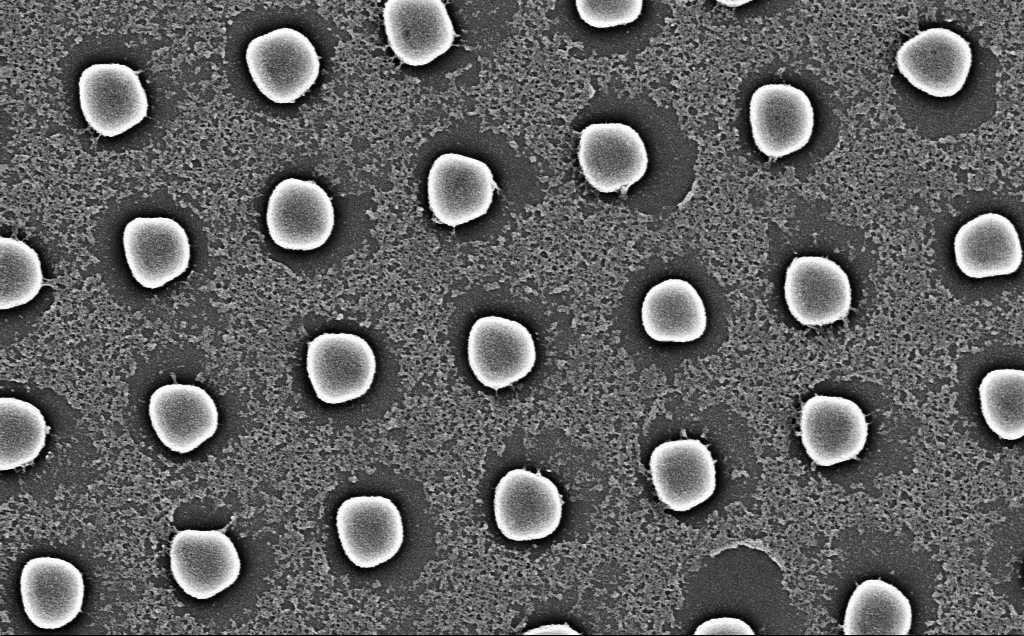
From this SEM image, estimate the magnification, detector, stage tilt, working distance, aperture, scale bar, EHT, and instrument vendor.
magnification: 39.67 K X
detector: InLens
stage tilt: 0°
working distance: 7 mm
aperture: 30 µm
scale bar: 1000 nm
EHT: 5 kV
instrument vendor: Zeiss